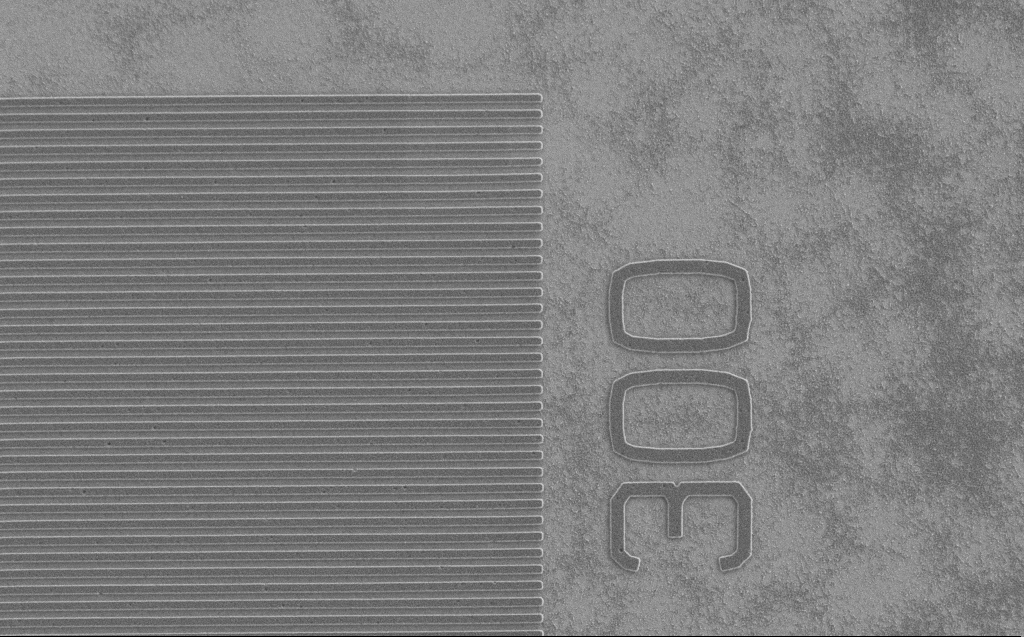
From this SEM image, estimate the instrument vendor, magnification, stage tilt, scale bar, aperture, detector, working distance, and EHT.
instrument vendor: Zeiss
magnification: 10.06 K X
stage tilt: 0°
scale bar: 2000 nm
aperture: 30 µm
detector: SE2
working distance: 5 mm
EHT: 1.2 kV